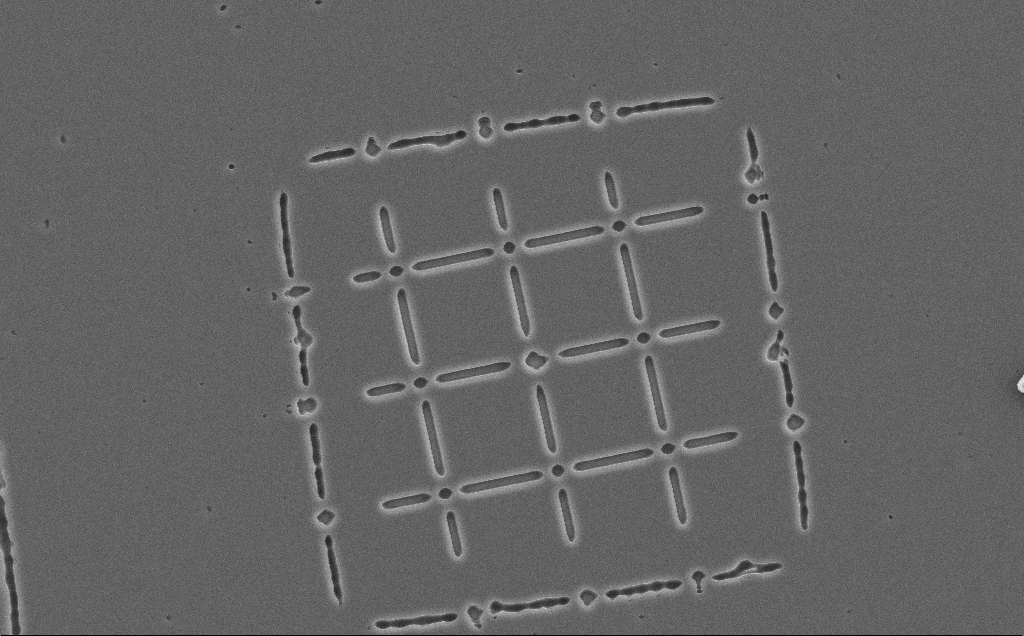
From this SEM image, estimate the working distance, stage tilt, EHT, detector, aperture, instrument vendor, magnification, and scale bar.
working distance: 12 mm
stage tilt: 0°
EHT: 10 kV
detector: SE2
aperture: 30 µm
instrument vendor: Zeiss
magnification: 2.07 K X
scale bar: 10000 nm